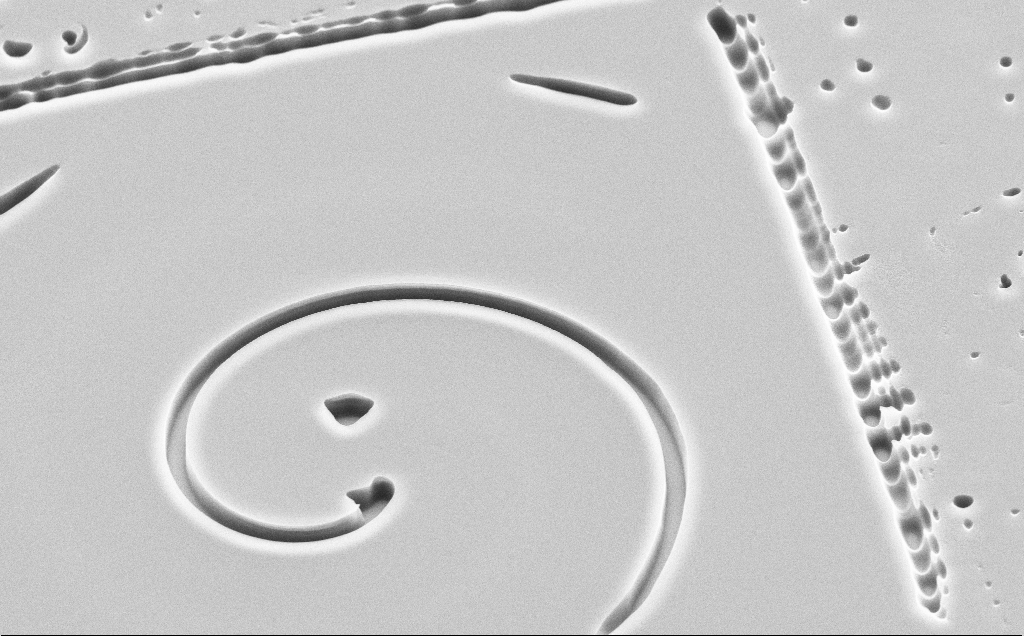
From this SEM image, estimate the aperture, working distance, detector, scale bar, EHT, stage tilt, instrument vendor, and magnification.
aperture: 30 µm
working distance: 11 mm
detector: SE2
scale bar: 10000 nm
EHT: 10 kV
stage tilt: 45°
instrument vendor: Zeiss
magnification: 4.42 K X